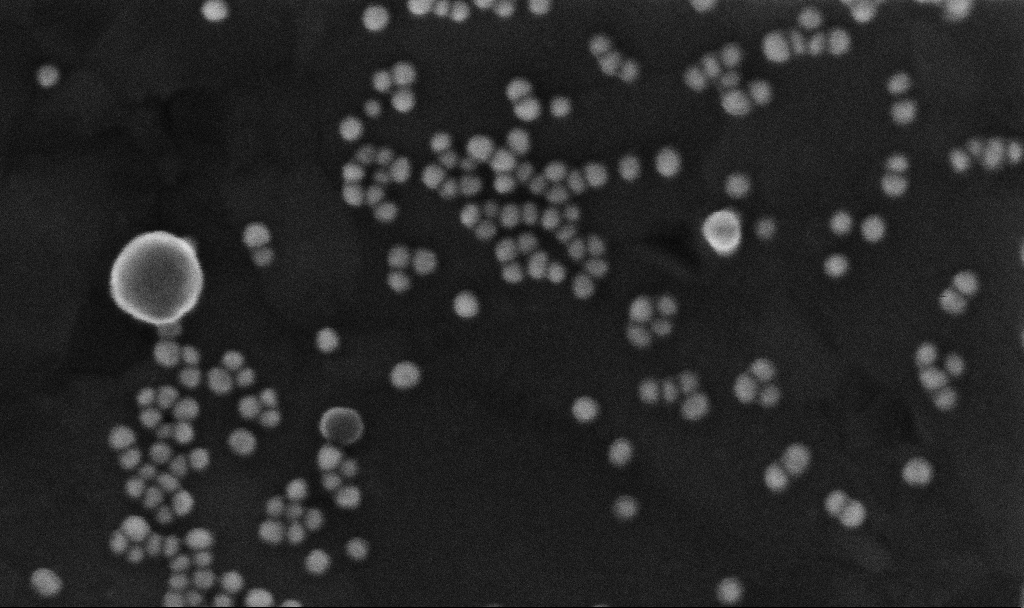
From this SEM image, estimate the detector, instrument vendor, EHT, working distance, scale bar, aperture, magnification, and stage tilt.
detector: InLens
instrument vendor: Zeiss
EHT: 10 kV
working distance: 3.7 mm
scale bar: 100 nm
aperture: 30 µm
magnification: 448.47 K X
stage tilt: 0°